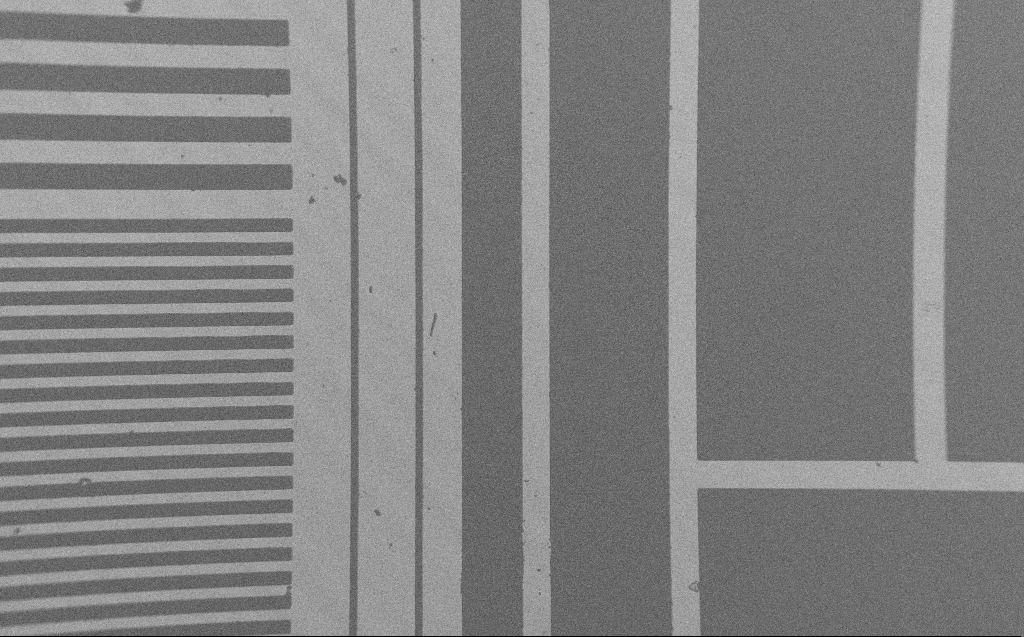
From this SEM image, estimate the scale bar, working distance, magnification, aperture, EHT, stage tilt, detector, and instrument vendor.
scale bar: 200000 nm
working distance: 4 mm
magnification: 0.216 K X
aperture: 30 µm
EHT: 1.2 kV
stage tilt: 0°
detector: SE2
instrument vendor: Zeiss